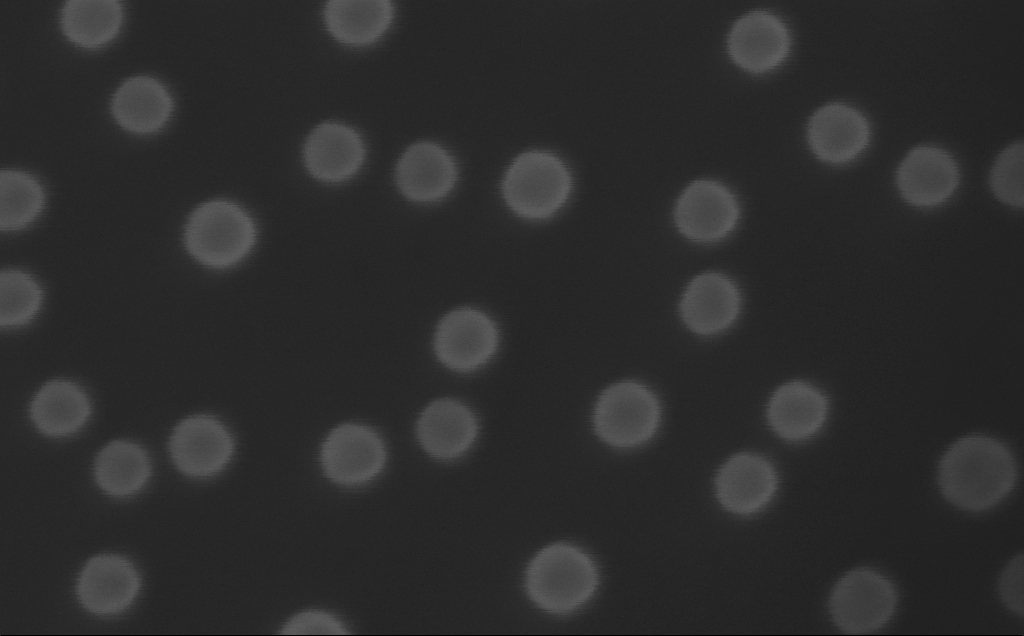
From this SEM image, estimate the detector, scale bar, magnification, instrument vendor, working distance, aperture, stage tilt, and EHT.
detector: InLens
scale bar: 20 nm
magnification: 800 K X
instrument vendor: Zeiss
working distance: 4 mm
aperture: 30 µm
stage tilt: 0°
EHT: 10 kV